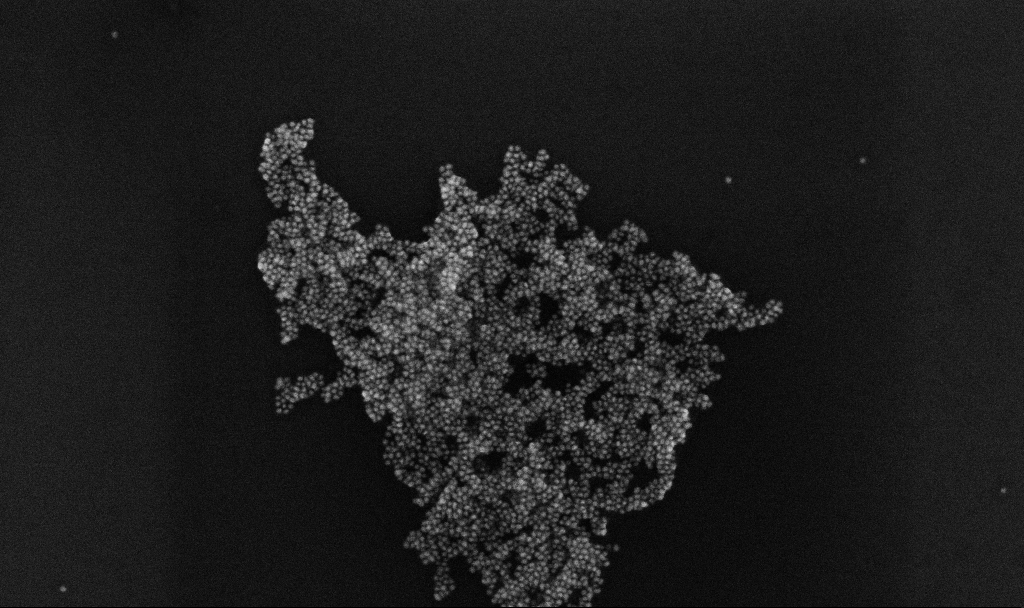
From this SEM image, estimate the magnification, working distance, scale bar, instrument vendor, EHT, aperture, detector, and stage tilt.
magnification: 100 K X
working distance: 3.3 mm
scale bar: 200 nm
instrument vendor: Zeiss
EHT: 10 kV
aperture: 30 µm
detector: InLens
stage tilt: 0°